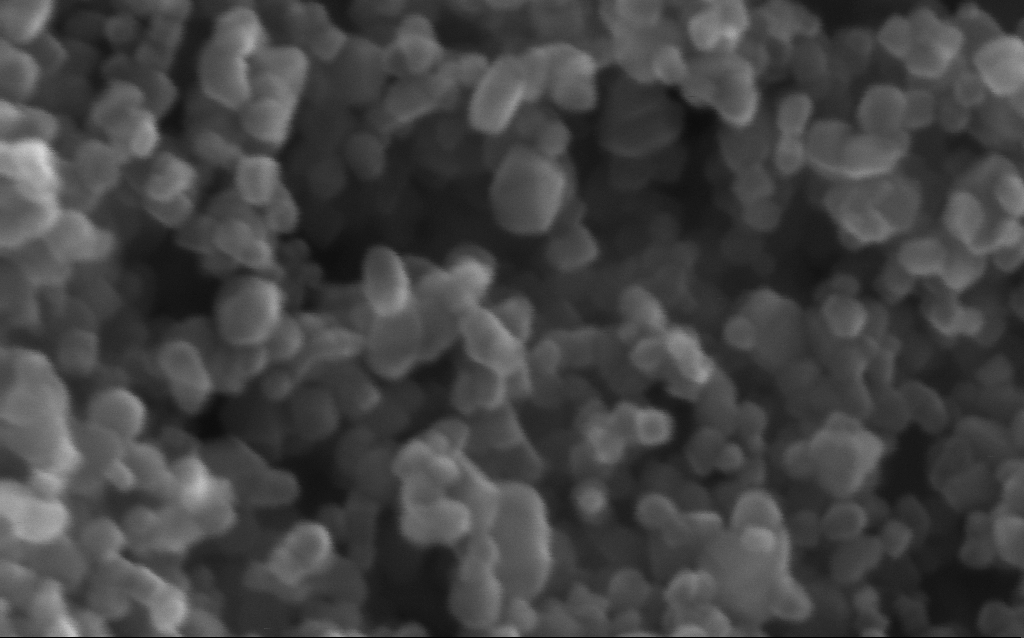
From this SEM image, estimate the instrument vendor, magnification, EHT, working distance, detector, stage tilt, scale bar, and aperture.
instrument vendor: Zeiss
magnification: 716 K X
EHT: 10 kV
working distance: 3 mm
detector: InLens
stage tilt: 0°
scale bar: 100 nm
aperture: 30 µm